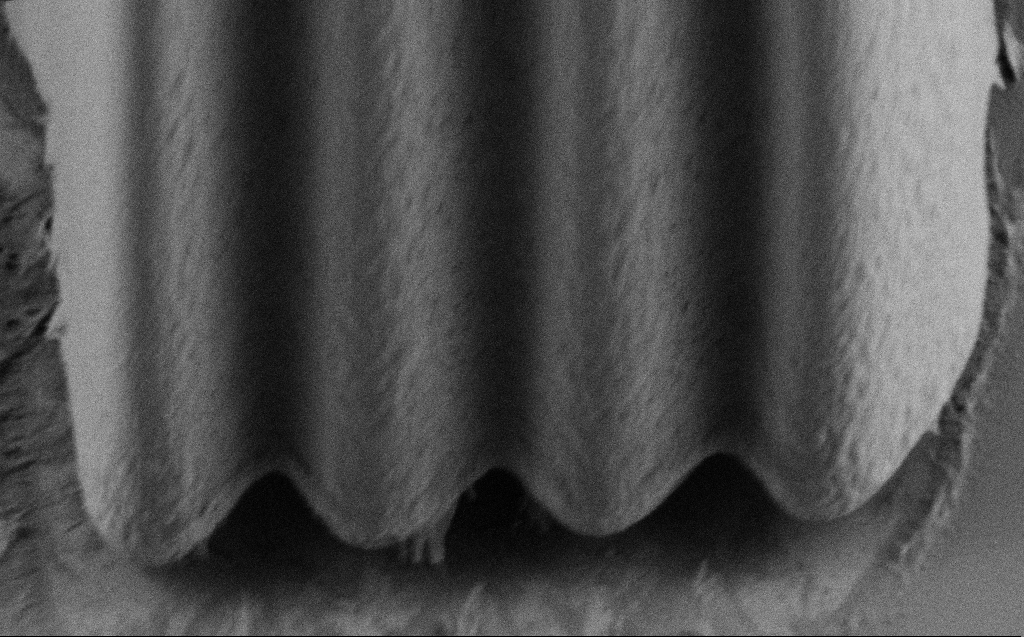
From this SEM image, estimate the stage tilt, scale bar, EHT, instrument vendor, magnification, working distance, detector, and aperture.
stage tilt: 45°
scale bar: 2000 nm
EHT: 0.9 kV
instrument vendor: Zeiss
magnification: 7.51 K X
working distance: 4 mm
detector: SE2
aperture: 30 µm